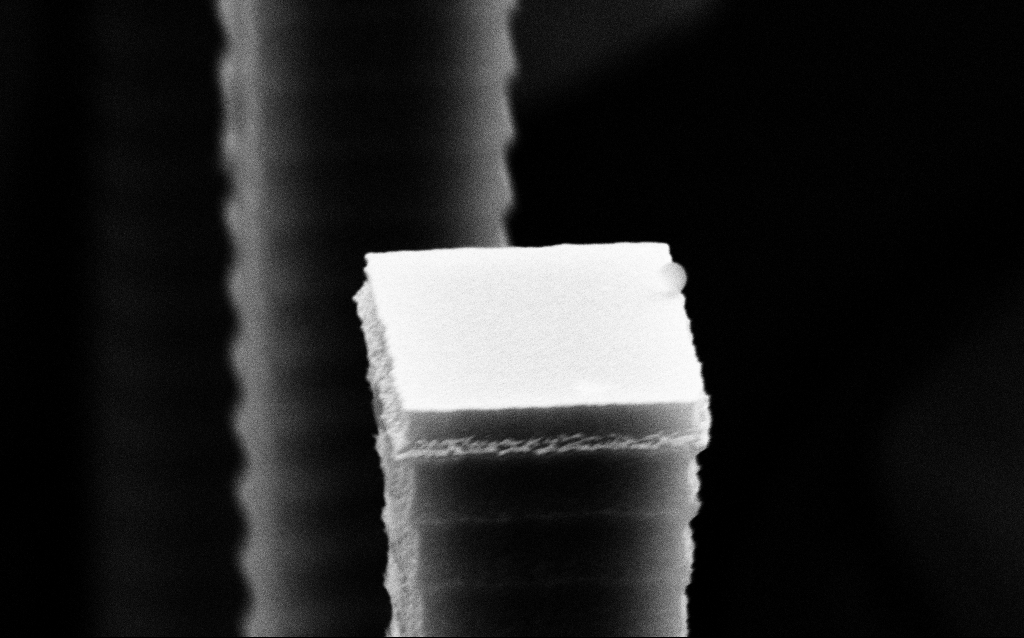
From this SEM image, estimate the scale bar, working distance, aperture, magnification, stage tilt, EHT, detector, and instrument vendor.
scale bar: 1000 nm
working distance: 6.5 mm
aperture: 30 µm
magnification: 63.87 K X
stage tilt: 70°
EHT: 8 kV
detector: SE2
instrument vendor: Zeiss